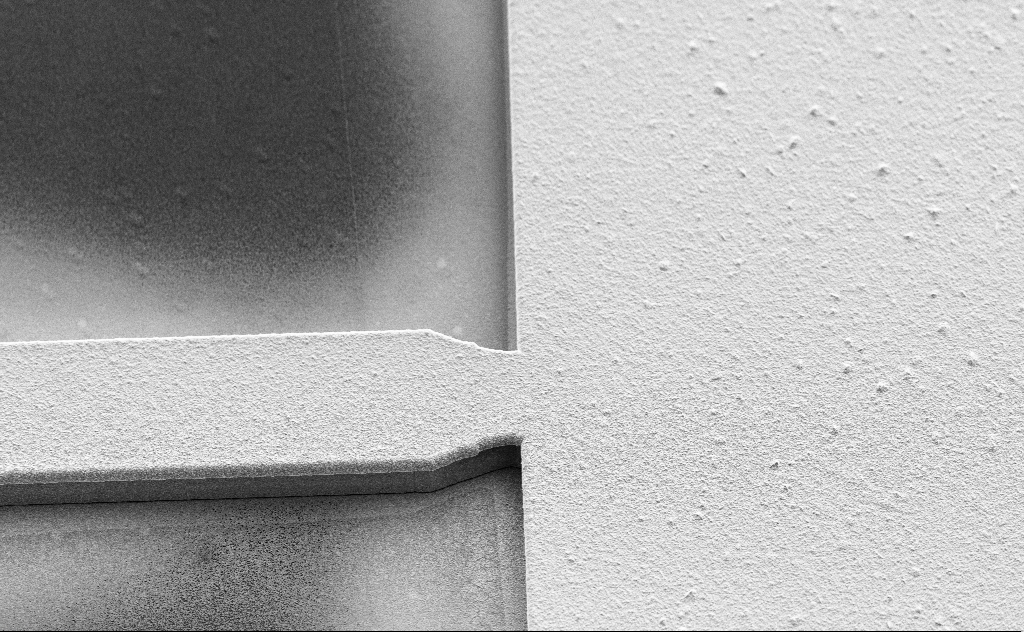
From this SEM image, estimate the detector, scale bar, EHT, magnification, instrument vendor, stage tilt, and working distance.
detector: SE2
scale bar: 10000 nm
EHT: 5 kV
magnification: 4.72 K X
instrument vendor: Zeiss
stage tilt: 45°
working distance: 10 mm